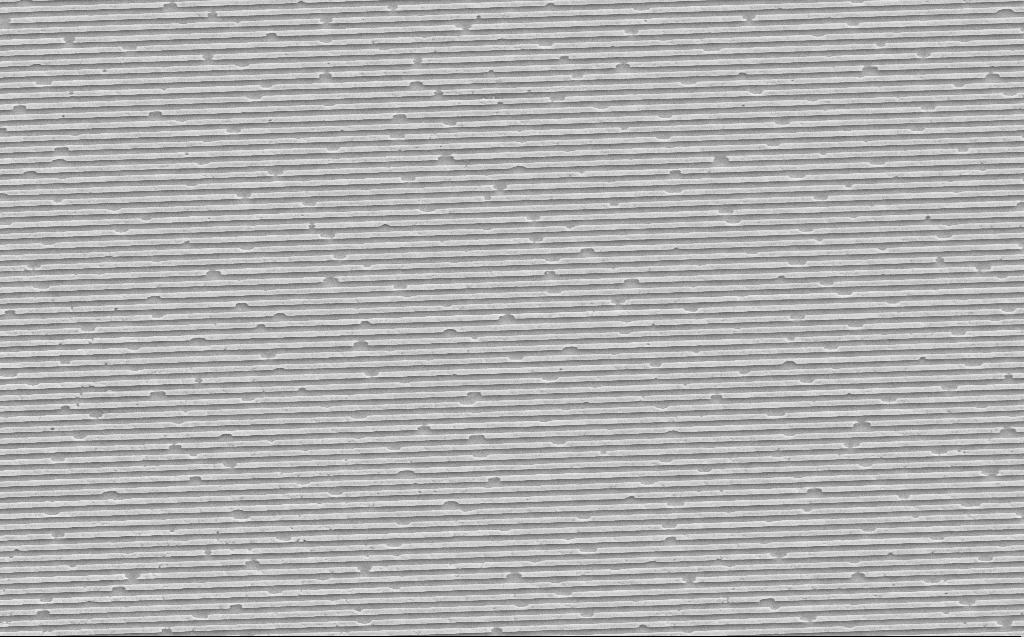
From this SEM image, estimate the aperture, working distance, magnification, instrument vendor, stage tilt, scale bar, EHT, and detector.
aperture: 30 µm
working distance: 10 mm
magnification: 8 K X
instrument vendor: Zeiss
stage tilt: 0°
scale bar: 2000 nm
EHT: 10 kV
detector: SE2